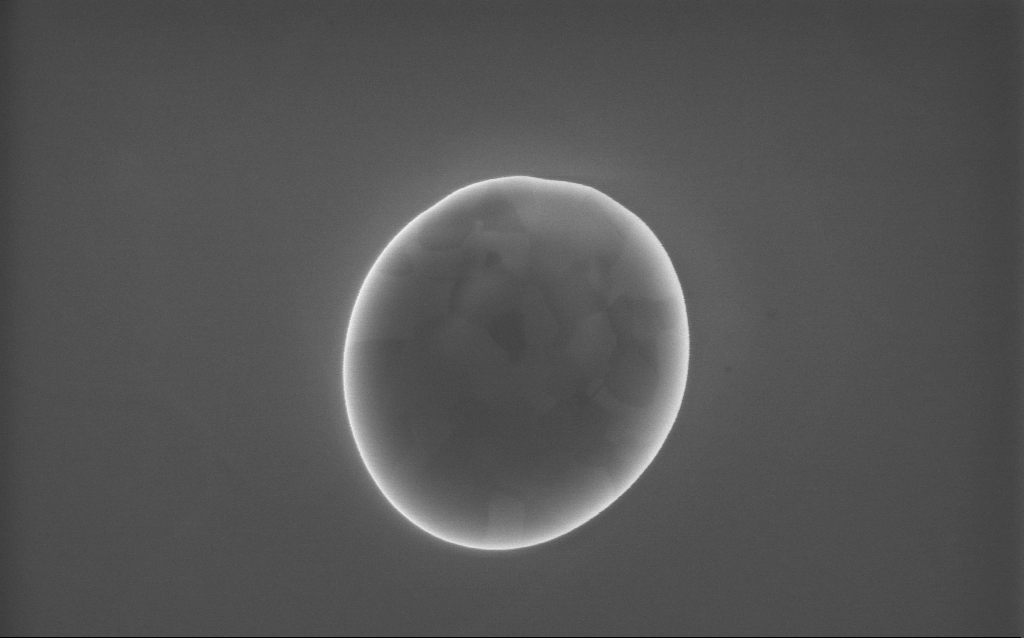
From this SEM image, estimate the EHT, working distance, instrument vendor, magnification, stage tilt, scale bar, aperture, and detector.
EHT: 10 kV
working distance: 2 mm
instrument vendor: Zeiss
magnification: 70 K X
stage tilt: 0°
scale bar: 1000 nm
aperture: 30 µm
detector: InLens